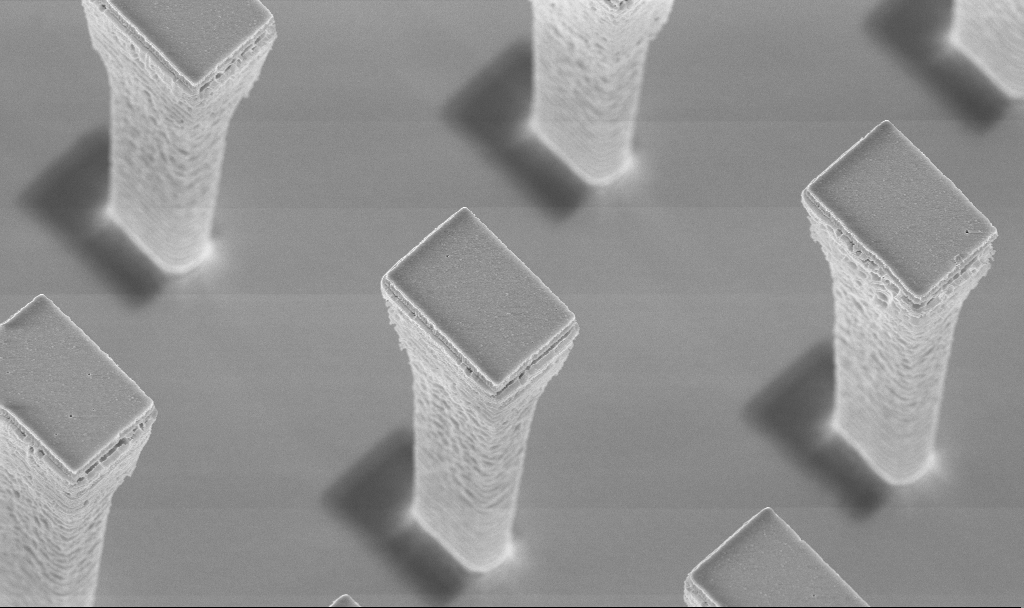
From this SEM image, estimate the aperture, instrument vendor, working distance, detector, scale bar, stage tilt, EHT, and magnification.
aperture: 30 µm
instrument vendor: Zeiss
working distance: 4.2 mm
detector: InLens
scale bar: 2000 nm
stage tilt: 20°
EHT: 5 kV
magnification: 13.53 K X